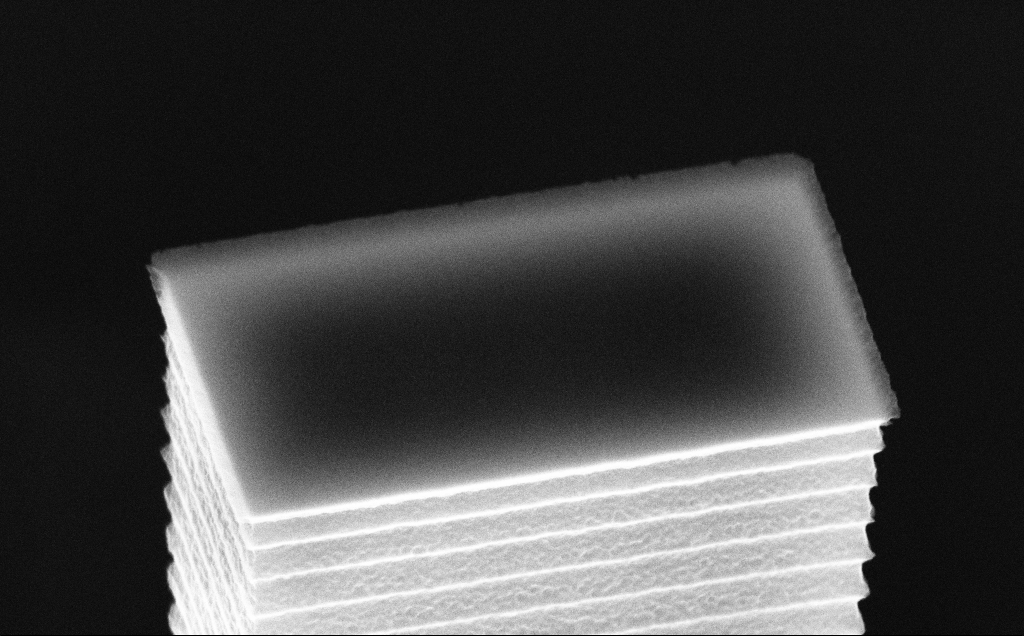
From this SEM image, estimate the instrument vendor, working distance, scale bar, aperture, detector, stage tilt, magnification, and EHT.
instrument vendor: Zeiss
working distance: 7 mm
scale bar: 1000 nm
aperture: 30 µm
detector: InLens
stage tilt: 45°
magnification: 52.02 K X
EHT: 10 kV